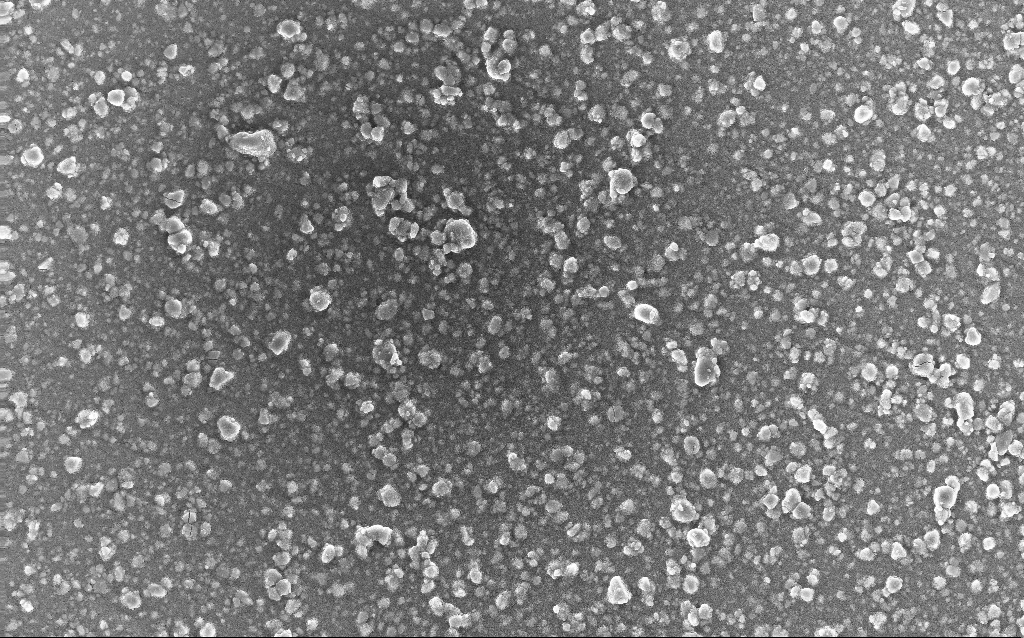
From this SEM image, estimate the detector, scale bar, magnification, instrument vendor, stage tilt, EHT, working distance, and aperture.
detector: InLens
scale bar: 20000 nm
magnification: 0.77 K X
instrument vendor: Zeiss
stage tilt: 0°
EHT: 20 kV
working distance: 3 mm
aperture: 30 µm